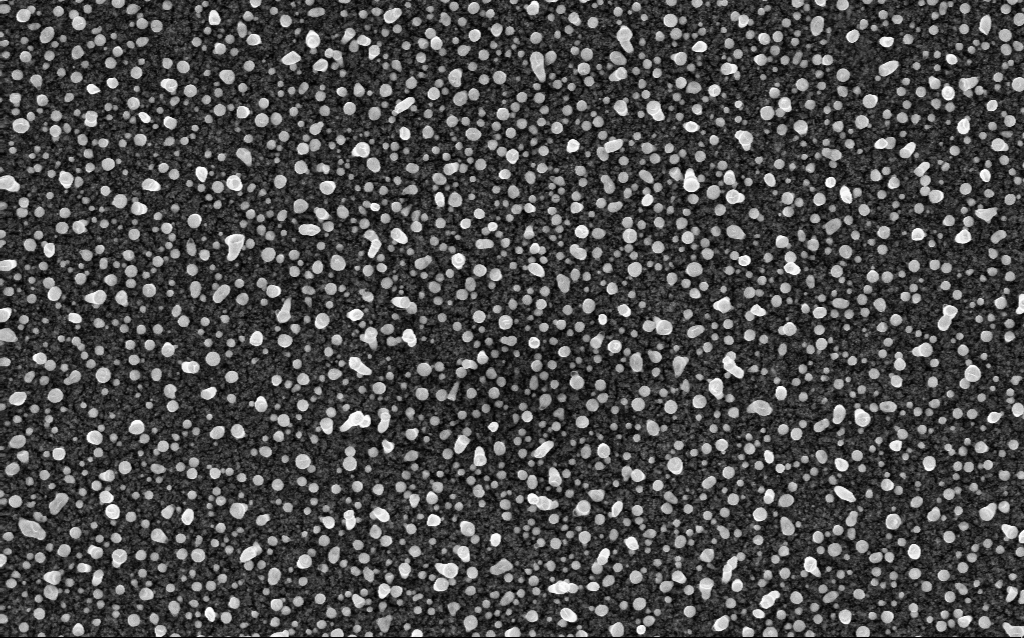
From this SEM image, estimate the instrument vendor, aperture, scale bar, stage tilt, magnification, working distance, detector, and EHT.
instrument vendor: Zeiss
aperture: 30 µm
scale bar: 1000 nm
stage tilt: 0°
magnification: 50 K X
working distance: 2.1 mm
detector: InLens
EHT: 5 kV